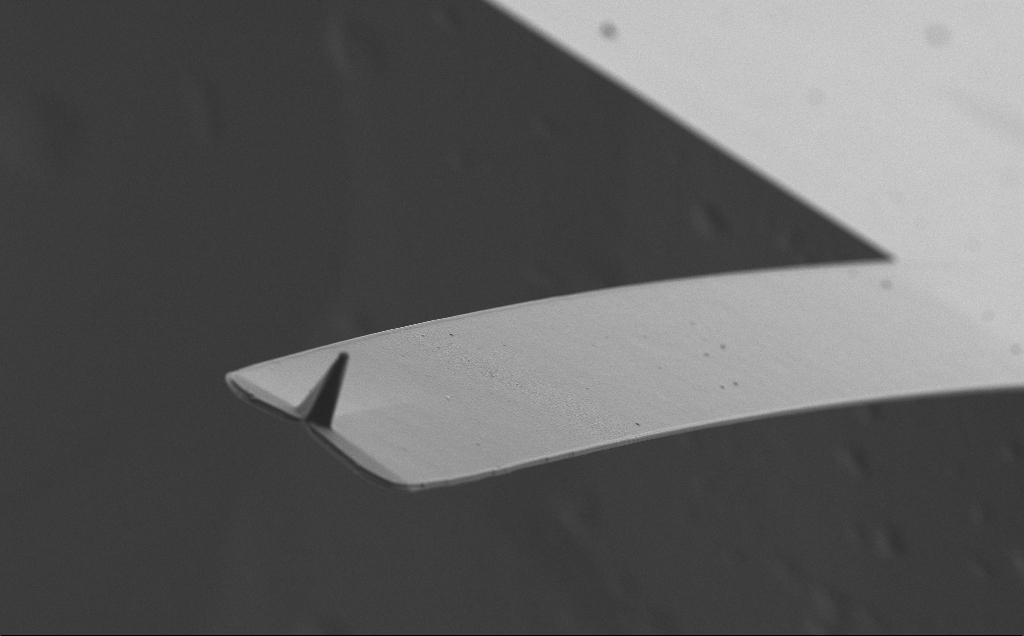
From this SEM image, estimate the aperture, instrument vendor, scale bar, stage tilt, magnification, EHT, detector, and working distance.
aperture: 30 µm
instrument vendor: Zeiss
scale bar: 20000 nm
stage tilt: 30°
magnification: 2.38 K X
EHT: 5 kV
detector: SE2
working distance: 8 mm